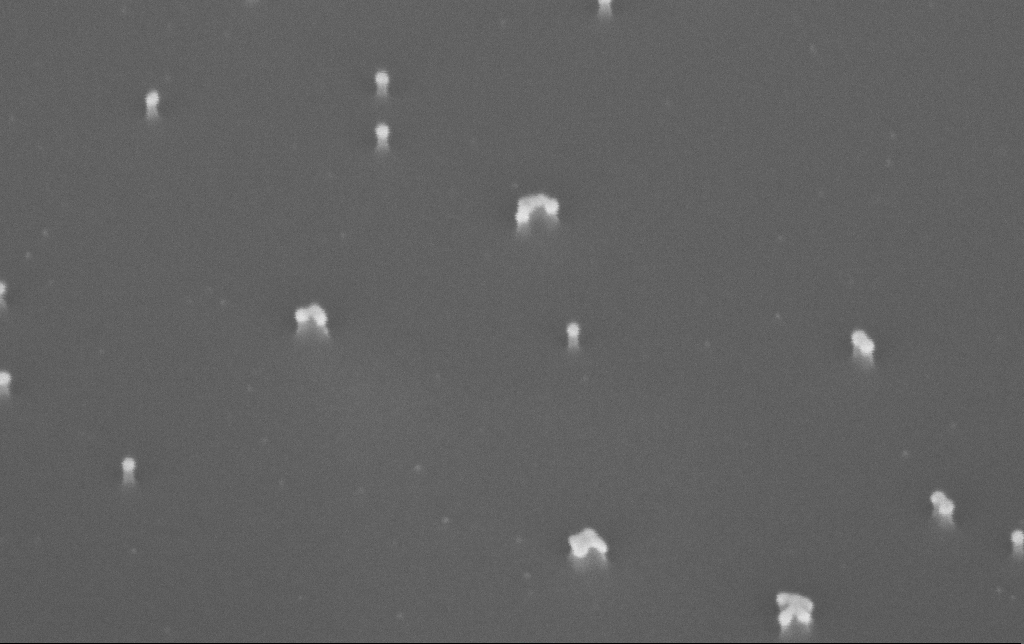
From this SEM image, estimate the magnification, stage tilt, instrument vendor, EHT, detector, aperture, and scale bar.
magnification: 300 K X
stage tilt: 45°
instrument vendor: Zeiss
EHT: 10 kV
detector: InLens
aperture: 30 µm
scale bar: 200 nm